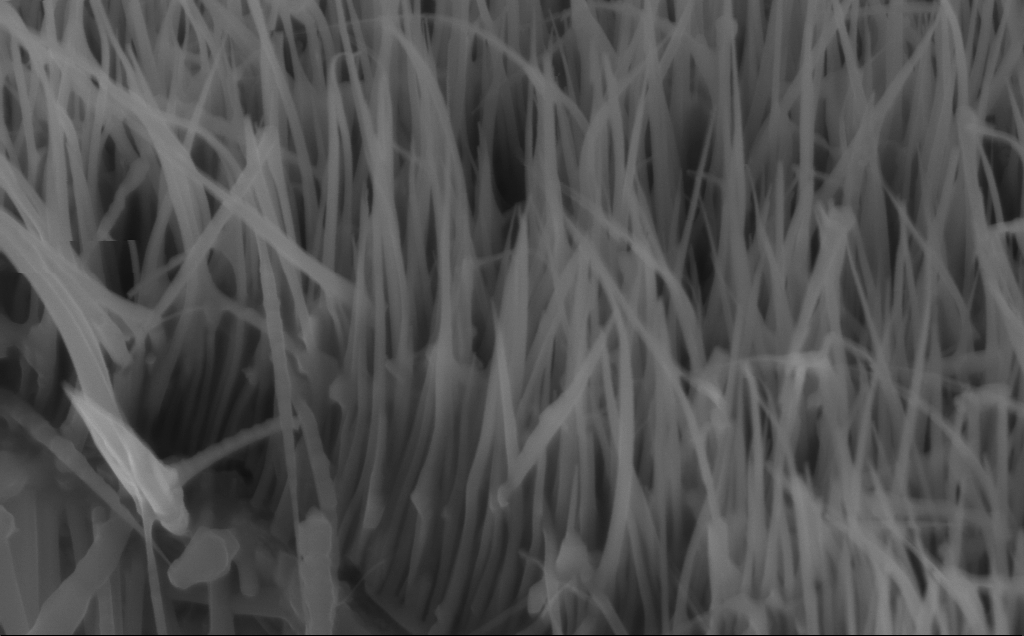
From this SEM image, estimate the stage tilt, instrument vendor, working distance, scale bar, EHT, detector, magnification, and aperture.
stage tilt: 45°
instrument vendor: Zeiss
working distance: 5 mm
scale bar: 200 nm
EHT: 10 kV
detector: InLens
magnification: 80 K X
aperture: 30 µm